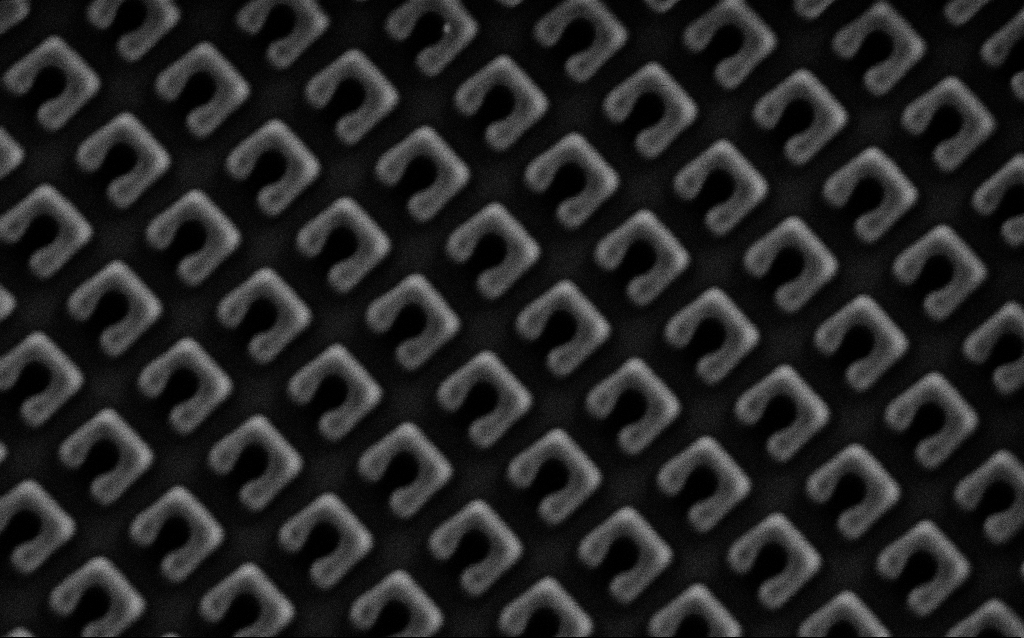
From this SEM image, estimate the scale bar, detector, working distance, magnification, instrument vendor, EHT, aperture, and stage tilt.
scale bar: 200 nm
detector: SE2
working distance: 7.9 mm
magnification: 83.3 K X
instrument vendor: Zeiss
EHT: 1.5 kV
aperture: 30 µm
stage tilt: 0°